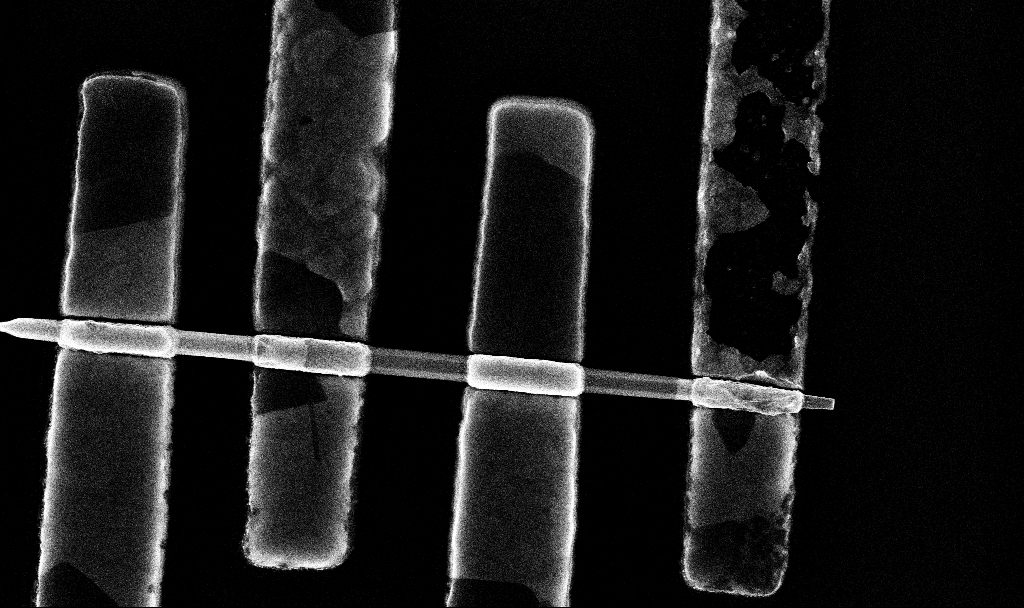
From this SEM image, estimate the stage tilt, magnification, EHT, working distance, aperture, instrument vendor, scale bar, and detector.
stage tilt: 0°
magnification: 68.5 K X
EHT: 10 kV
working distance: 6.8 mm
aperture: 30 µm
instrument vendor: Zeiss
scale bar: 1000 nm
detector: InLens